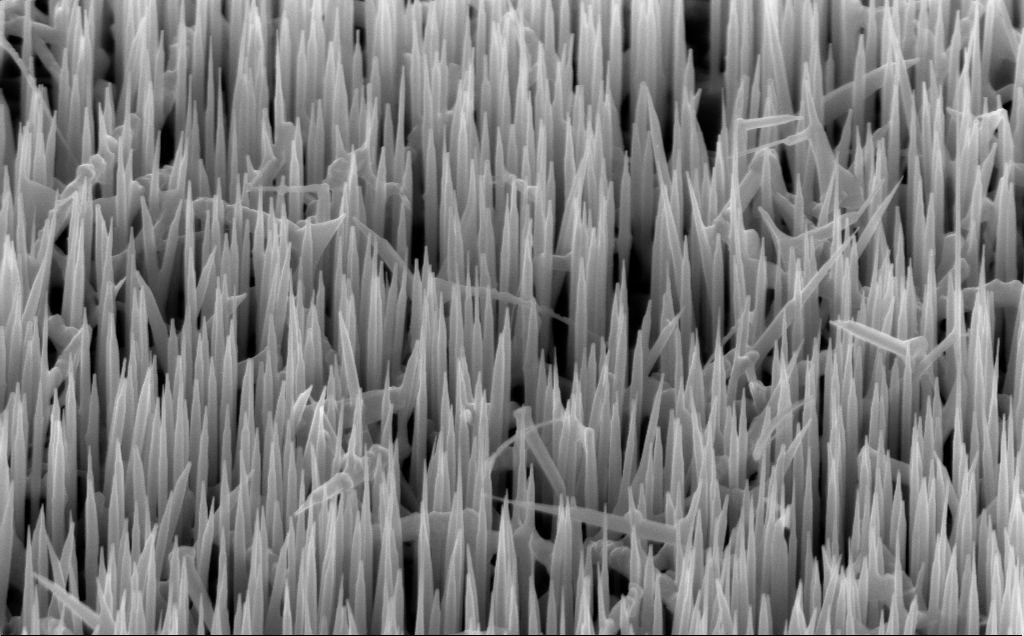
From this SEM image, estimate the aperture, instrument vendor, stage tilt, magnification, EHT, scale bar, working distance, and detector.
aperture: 30 µm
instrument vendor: Zeiss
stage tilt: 45°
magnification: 40 K X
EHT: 10 kV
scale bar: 1000 nm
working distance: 6 mm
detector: InLens